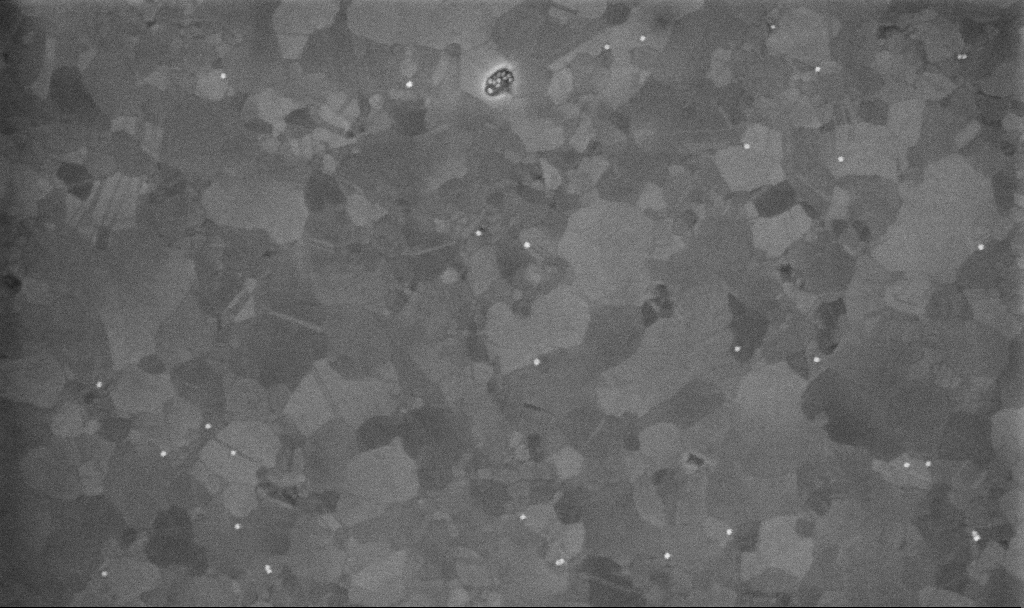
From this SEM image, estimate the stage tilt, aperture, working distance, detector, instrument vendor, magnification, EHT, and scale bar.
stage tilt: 0°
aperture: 30 µm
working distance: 7.1 mm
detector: InLens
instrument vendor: Zeiss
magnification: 100 K X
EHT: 10 kV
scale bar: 200 nm